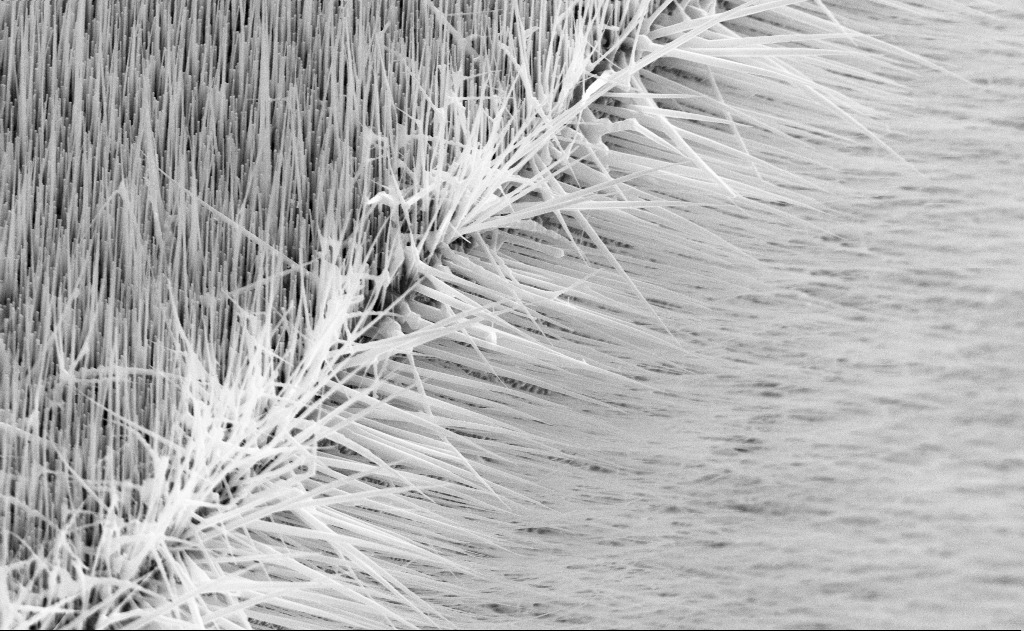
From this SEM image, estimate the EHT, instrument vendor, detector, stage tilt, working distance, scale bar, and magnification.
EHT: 10 kV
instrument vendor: Zeiss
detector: SE2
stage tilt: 45°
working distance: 16 mm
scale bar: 1000 nm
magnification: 20 K X